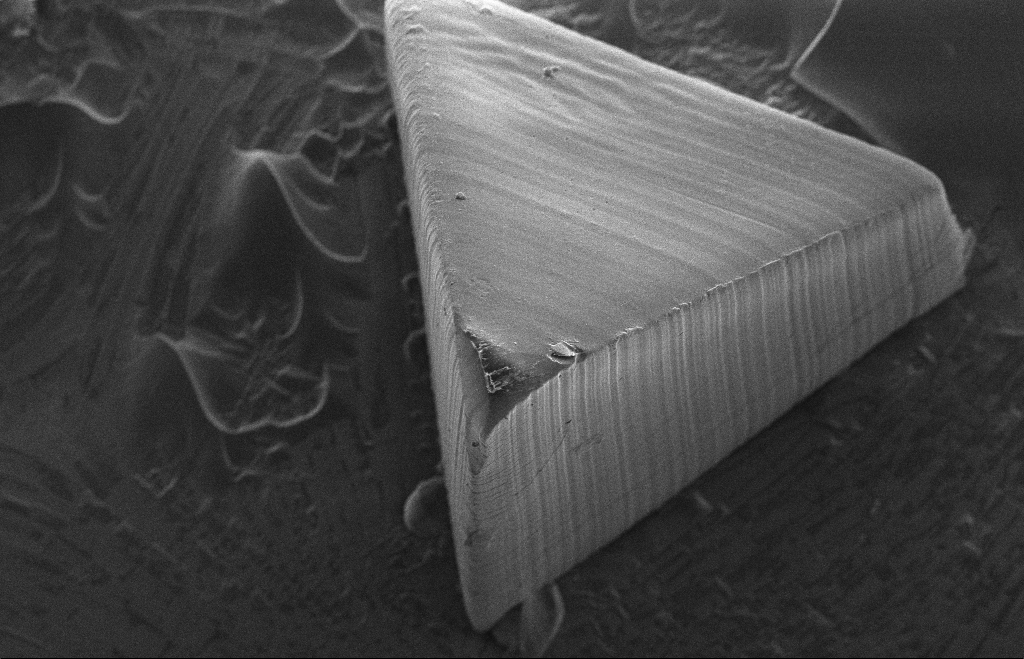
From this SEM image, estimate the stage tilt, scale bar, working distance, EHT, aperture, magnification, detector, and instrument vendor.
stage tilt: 17.3°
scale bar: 20000 nm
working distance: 14 mm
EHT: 5 kV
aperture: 30 µm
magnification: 0.751 K X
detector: SE2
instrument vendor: Zeiss